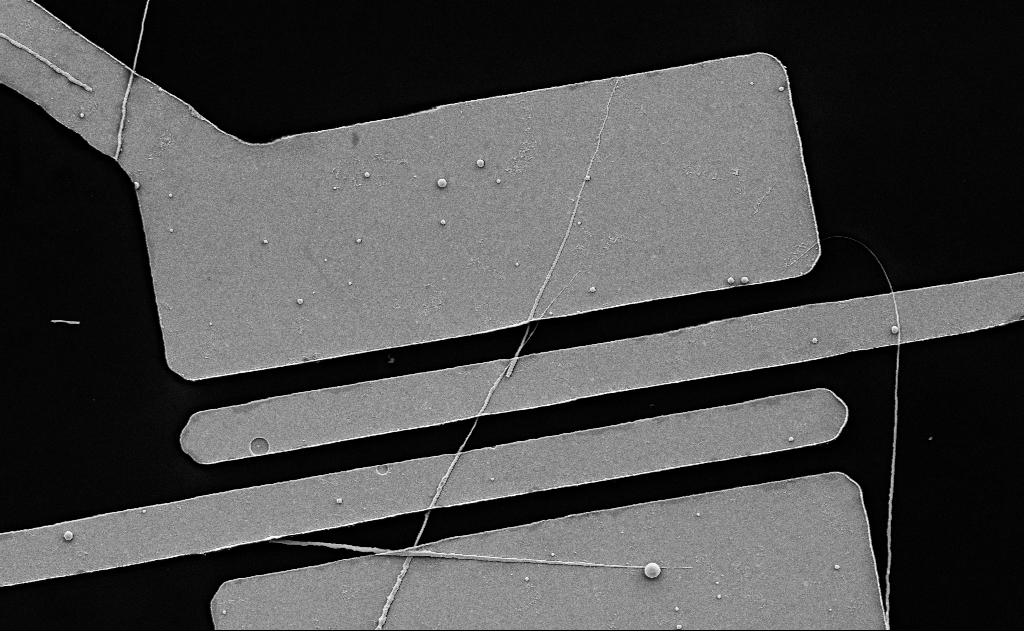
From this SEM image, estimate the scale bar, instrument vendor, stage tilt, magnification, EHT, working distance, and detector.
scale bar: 2000 nm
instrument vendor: Zeiss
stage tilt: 0°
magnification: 7.98 K X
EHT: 5 kV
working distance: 18 mm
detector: SE2